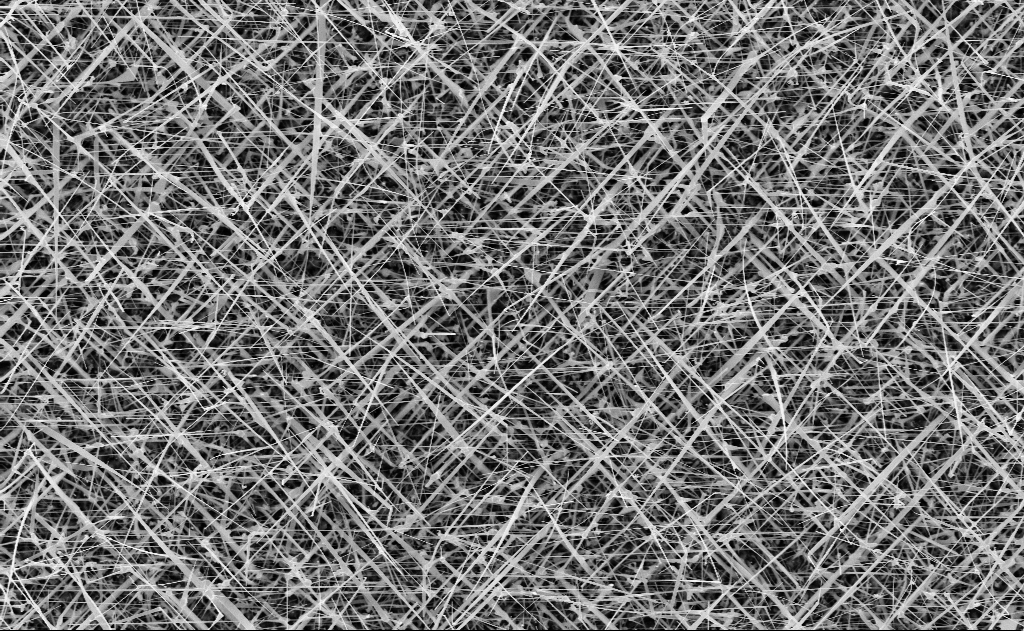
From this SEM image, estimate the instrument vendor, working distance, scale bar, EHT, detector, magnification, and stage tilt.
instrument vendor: Zeiss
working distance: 11 mm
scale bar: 2000 nm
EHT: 10 kV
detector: InLens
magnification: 10 K X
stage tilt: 0°